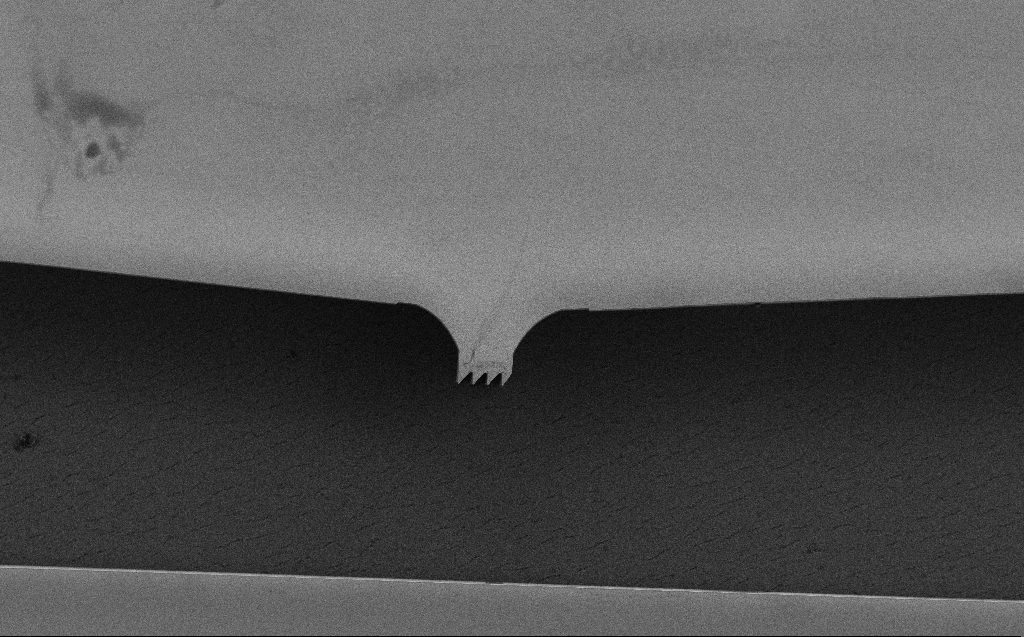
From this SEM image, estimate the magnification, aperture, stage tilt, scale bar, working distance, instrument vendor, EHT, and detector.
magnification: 0.478 K X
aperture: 30 µm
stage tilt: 0°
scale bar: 100000 nm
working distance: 5 mm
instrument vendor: Zeiss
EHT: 5 kV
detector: SE2